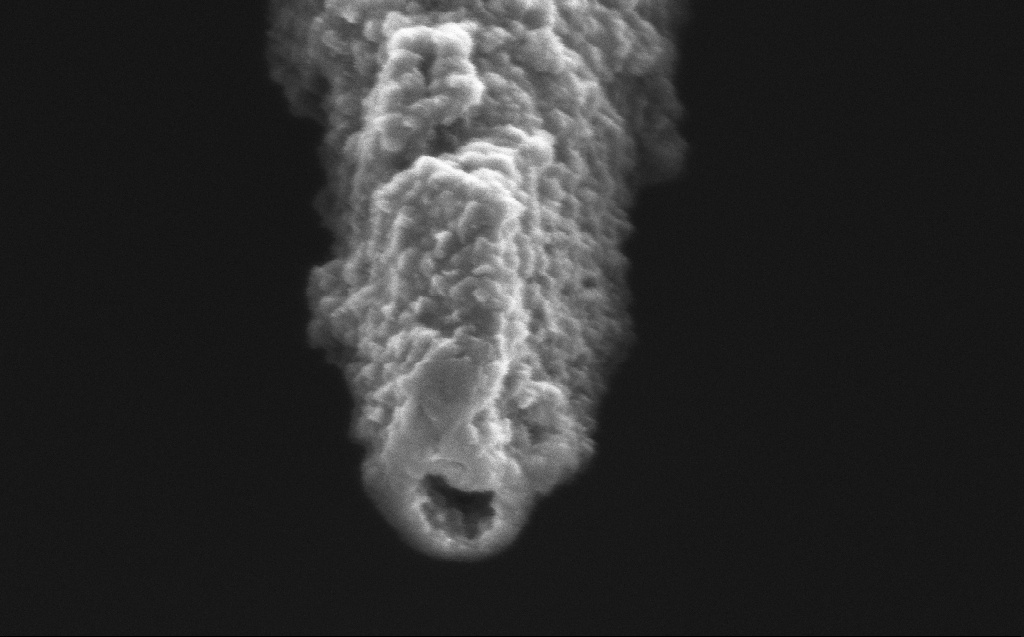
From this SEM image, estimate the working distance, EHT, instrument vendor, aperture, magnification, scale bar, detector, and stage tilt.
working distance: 3 mm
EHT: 2 kV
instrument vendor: Zeiss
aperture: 30 µm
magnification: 180 K X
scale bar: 100 nm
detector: InLens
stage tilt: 45°